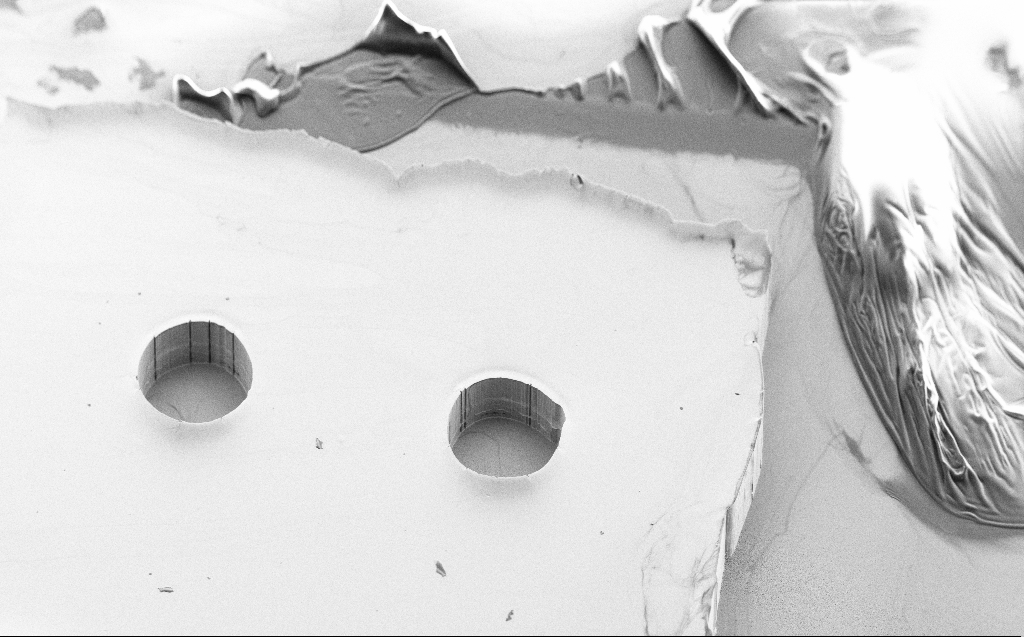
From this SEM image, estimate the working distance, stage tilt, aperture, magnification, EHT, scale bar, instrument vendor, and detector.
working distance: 6 mm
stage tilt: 45°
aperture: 30 µm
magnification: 0.099 K X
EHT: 10 kV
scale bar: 200000 nm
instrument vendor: Zeiss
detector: SE2